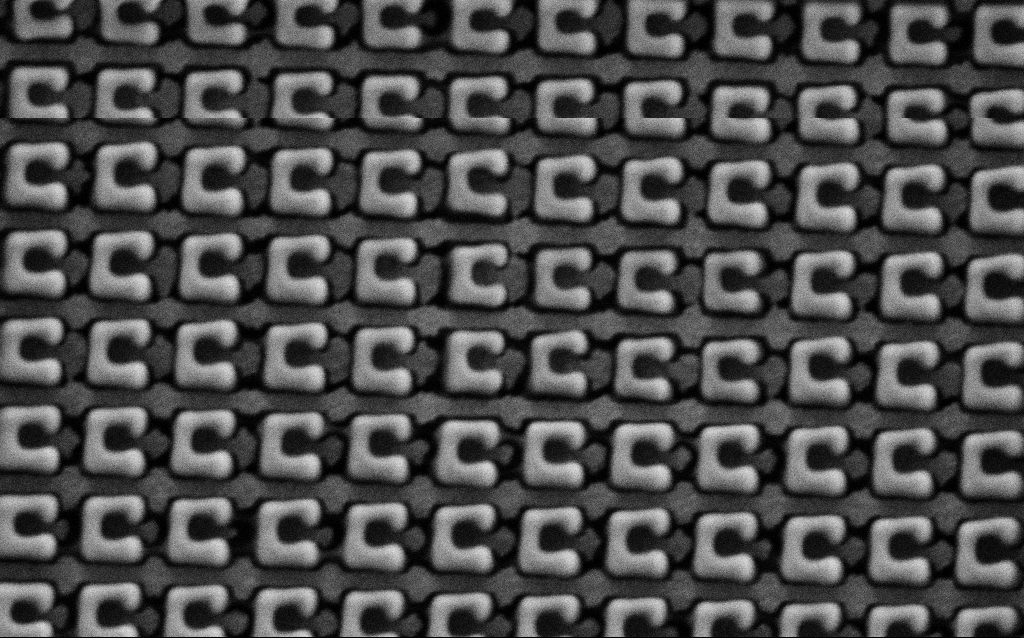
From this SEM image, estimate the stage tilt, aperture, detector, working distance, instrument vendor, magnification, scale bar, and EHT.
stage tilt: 0°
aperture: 30 µm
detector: SE2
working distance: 4.9 mm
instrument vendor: Zeiss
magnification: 69.73 K X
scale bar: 1000 nm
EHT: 1.5 kV